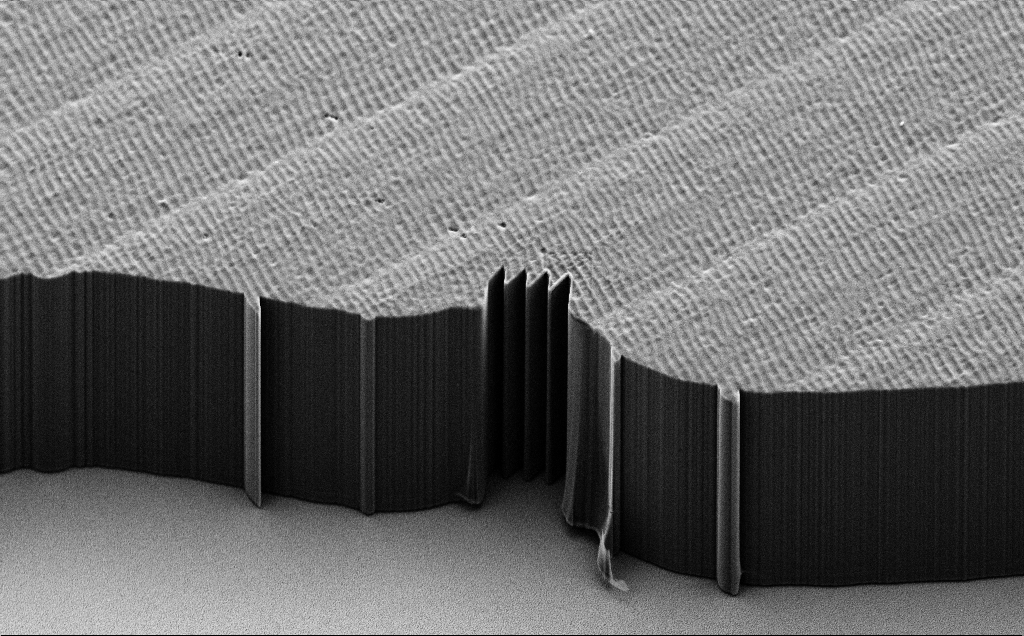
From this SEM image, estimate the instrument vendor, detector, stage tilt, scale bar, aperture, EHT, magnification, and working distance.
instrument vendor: Zeiss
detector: SE2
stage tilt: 45°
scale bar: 100000 nm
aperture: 30 µm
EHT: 10 kV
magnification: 0.707 K X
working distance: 8 mm